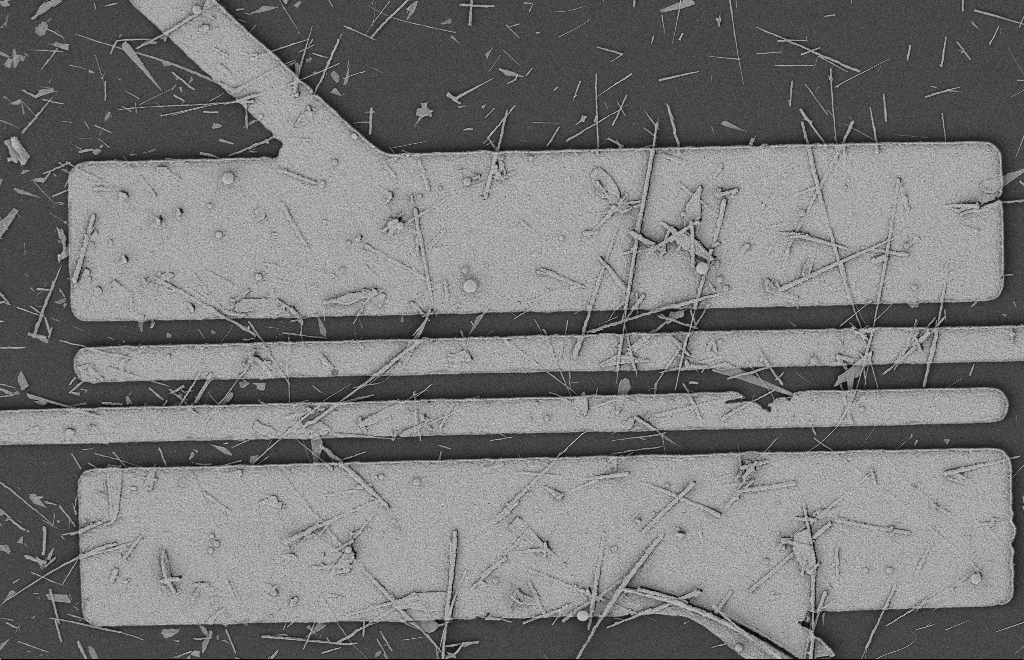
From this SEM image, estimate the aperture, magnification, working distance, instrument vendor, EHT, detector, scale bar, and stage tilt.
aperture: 20 µm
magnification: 5.58 K X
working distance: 12 mm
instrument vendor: Zeiss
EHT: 2 kV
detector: SE2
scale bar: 2000 nm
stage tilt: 0°